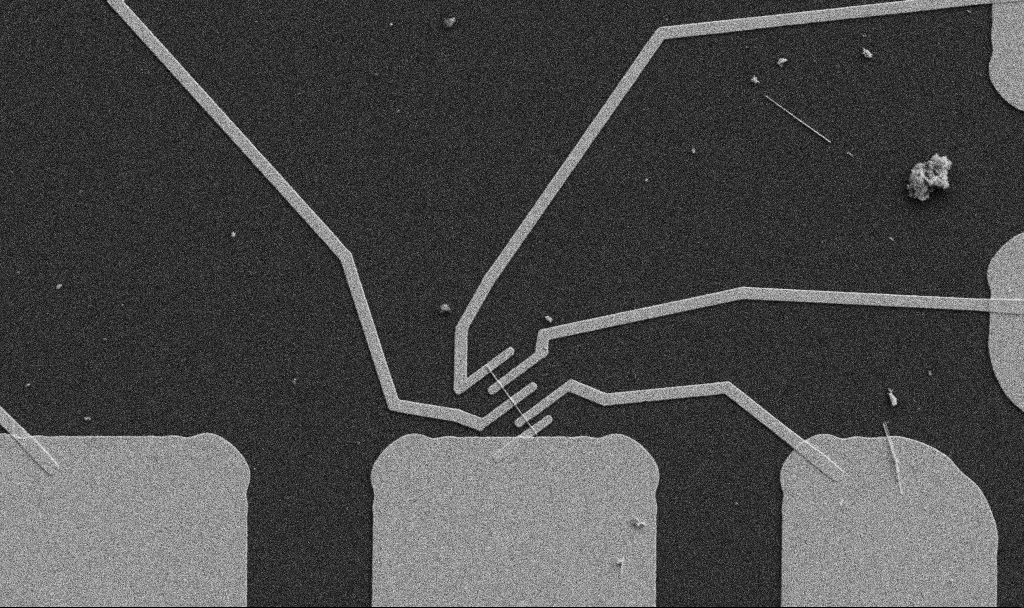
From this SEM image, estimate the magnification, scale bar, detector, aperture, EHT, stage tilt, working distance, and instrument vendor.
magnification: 5 K X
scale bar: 10000 nm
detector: SE2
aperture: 30 µm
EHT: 5 kV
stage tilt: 0°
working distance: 10.7 mm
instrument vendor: Zeiss